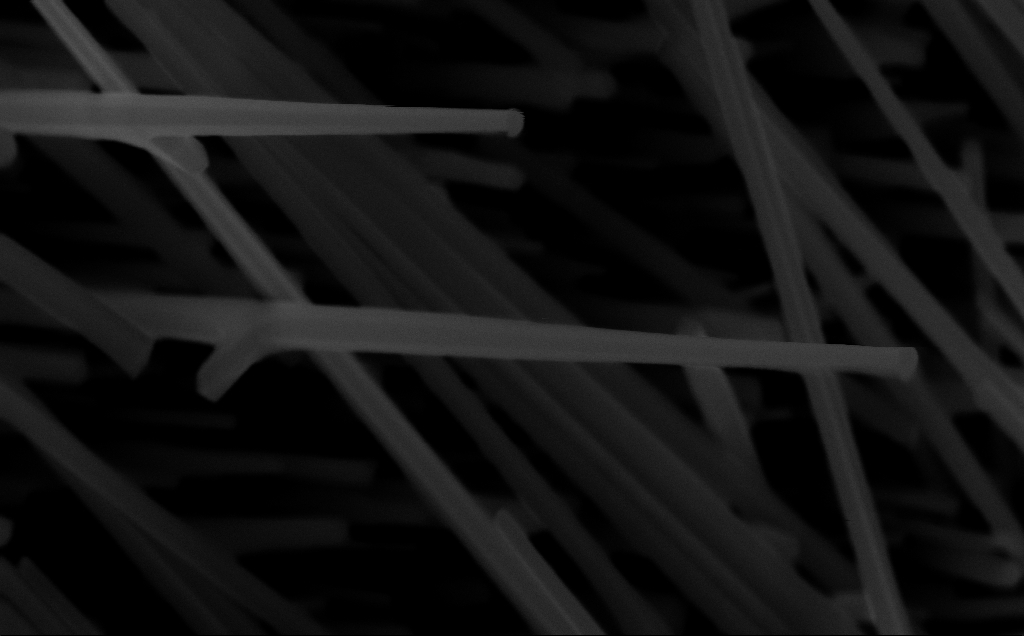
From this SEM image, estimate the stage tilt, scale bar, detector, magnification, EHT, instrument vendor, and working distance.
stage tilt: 0°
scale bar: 200 nm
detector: InLens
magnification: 81.77 K X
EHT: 10 kV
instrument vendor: Zeiss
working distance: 6 mm